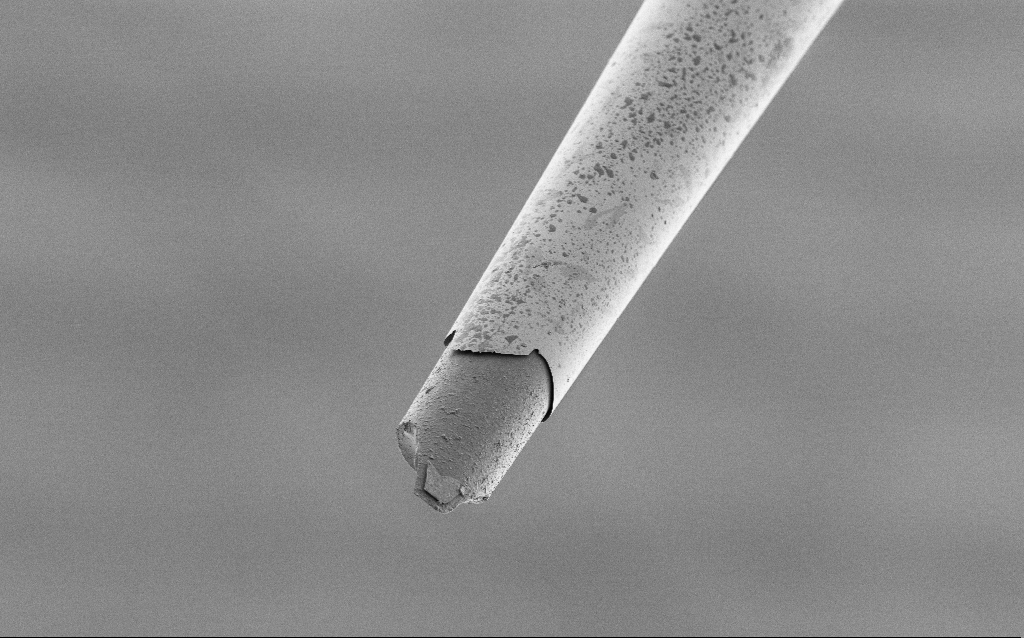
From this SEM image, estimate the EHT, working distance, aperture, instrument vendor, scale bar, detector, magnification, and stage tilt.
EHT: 1 kV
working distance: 7.7 mm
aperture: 30 µm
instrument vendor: Zeiss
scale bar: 20000 nm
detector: SE2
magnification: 1 K X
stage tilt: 45°